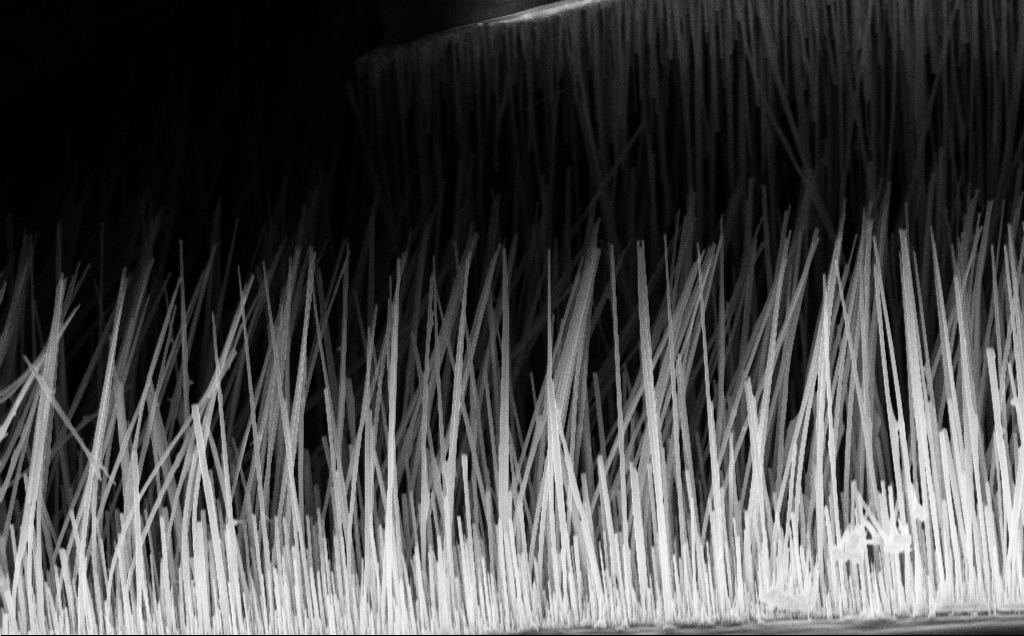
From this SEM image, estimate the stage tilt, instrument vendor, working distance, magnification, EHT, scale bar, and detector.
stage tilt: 44.7°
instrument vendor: Zeiss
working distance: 6 mm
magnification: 15.8 K X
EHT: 10 kV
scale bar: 1000 nm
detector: InLens